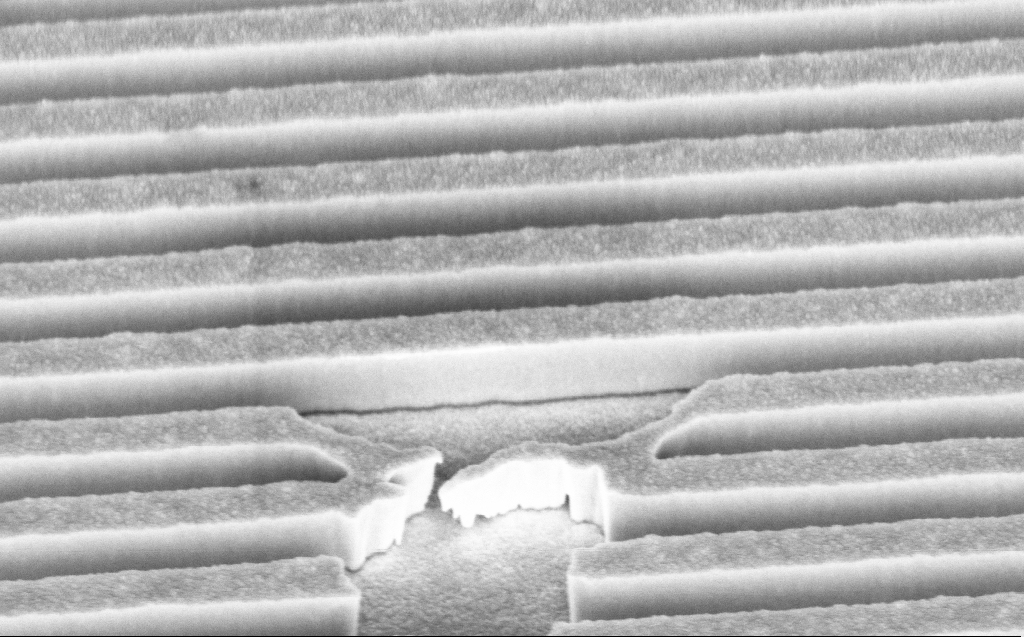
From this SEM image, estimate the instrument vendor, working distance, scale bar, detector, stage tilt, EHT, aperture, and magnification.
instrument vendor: Zeiss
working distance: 5 mm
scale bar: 1000 nm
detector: InLens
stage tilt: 45°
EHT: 5 kV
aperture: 30 µm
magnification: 58.98 K X